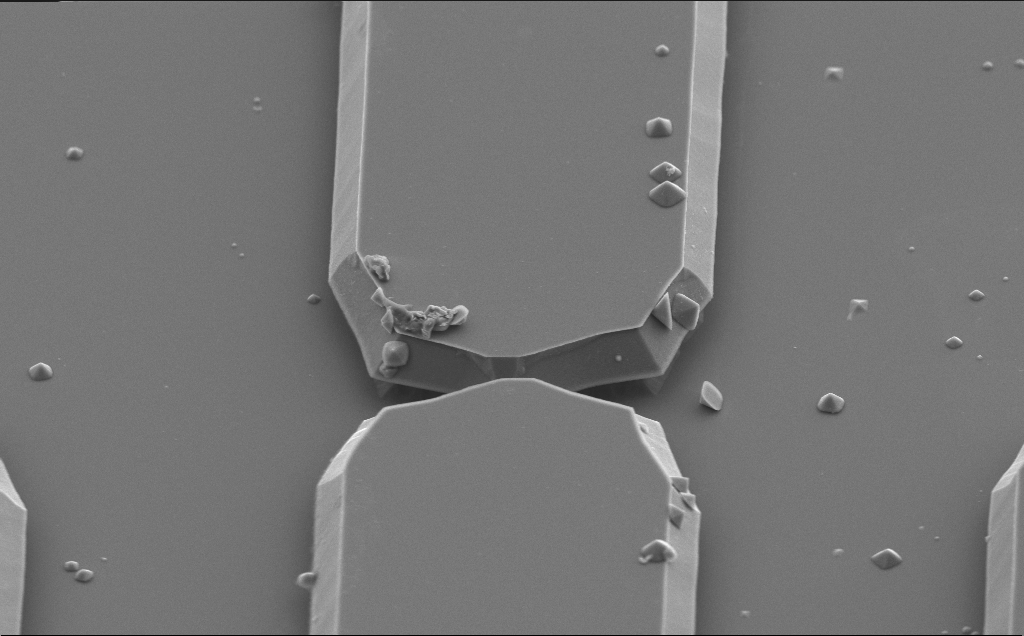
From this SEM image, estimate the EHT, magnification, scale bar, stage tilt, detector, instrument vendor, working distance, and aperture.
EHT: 5 kV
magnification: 6.21 K X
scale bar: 10000 nm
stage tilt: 50°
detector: SE2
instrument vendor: Zeiss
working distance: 10 mm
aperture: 30 µm